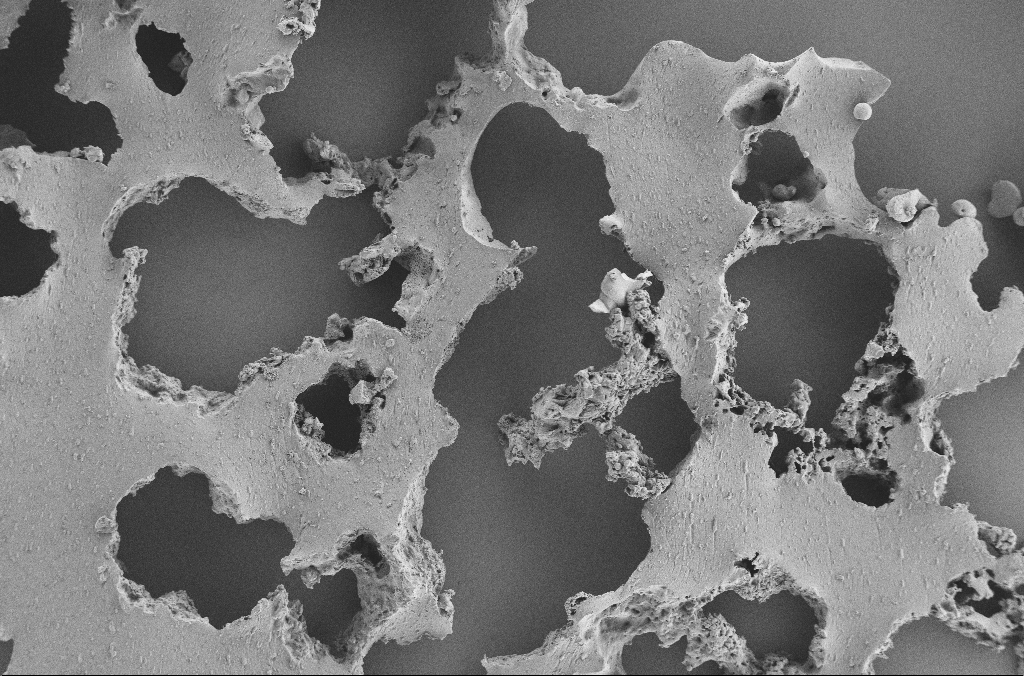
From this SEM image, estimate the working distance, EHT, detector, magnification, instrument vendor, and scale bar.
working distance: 3.6 mm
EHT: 2 kV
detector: SE2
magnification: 0.25 K X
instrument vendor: Zeiss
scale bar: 100000 nm